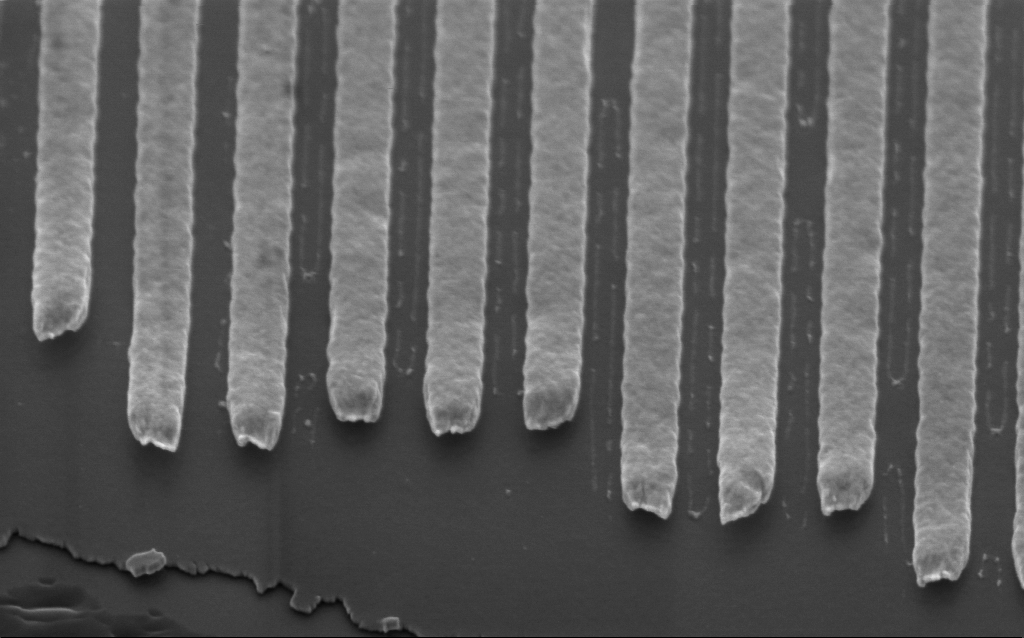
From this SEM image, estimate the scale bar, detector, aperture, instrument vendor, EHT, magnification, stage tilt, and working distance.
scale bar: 200 nm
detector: InLens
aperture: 30 µm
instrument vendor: Zeiss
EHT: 2 kV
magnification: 72.81 K X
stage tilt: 45°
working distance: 3.4 mm